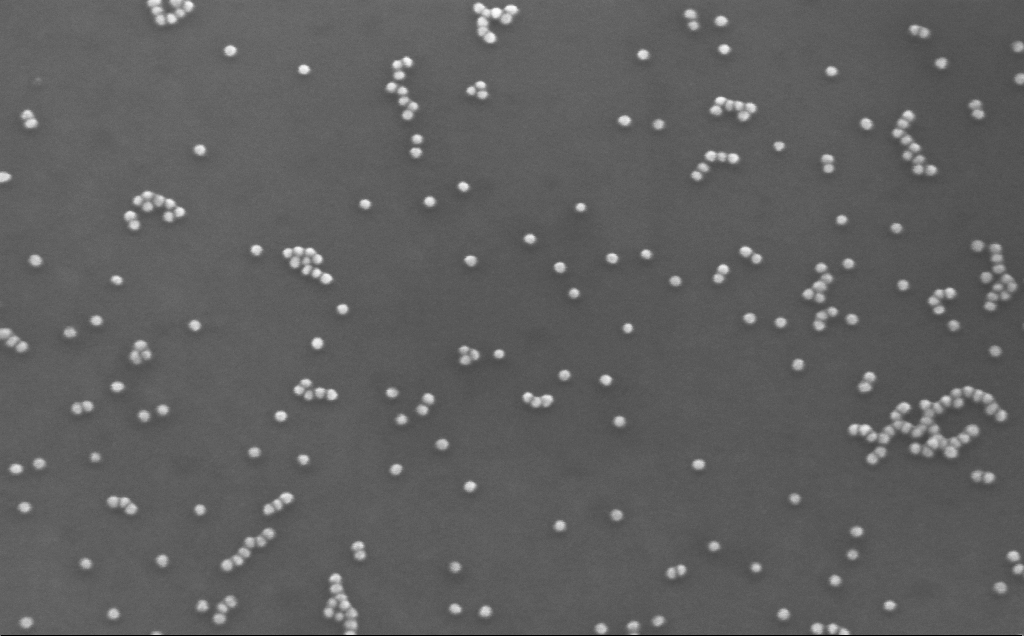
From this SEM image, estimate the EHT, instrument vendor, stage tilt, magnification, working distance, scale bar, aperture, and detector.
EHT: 10 kV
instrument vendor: Zeiss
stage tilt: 25°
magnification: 234.51 K X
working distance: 4 mm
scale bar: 100 nm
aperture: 30 µm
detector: InLens